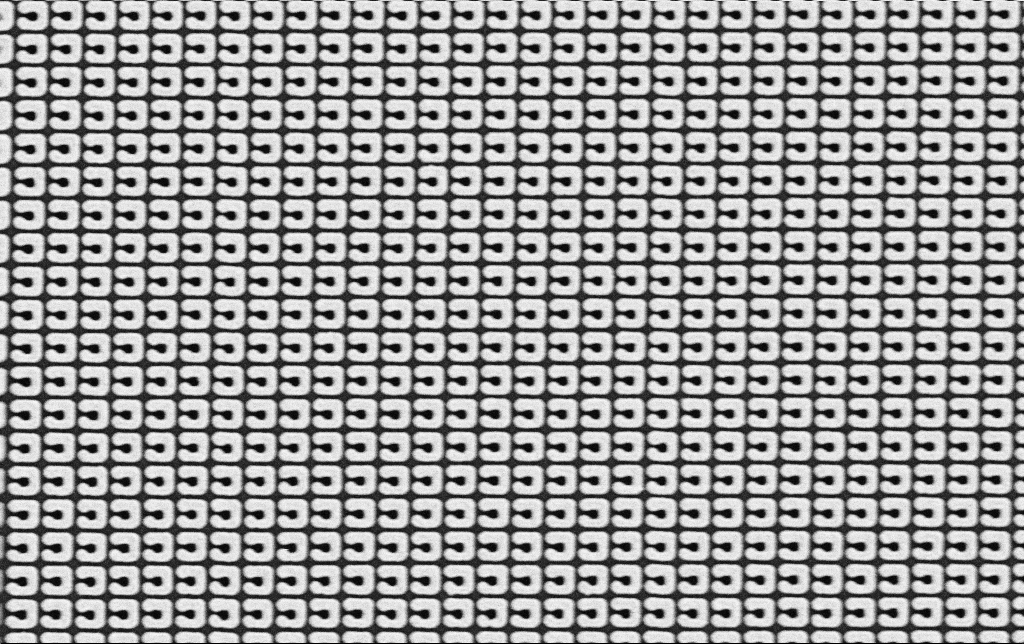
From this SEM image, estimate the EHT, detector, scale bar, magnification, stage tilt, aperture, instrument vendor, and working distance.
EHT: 3 kV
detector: SE2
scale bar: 2000 nm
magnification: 26.8 K X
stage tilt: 0°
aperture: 30 µm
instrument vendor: Zeiss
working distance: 5 mm